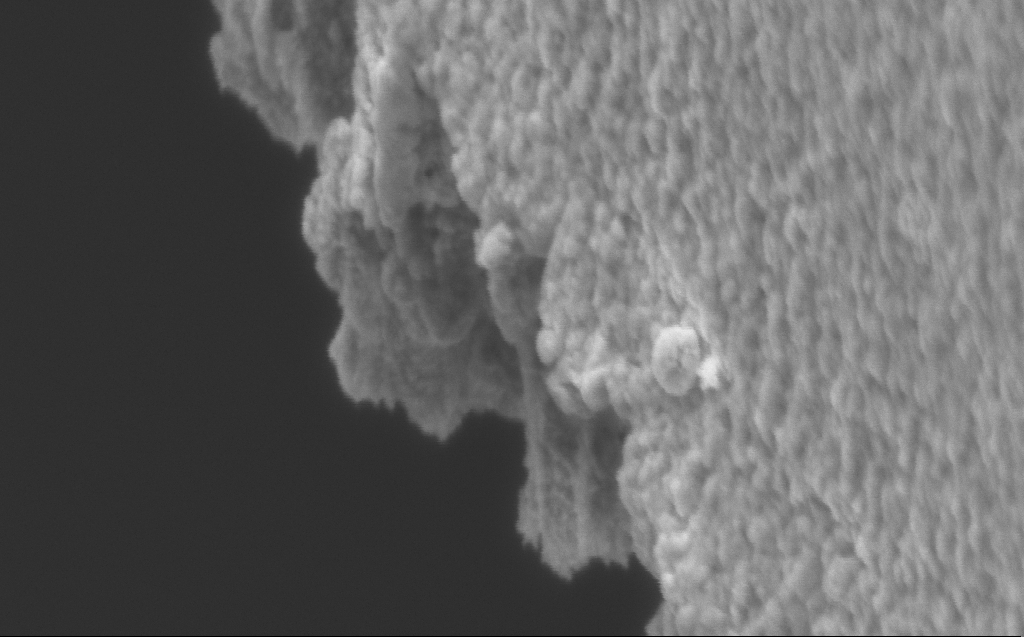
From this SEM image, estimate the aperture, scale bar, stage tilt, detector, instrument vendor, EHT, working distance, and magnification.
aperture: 30 µm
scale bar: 200 nm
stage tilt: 45°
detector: InLens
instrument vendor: Zeiss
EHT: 3 kV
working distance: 8 mm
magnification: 115.02 K X